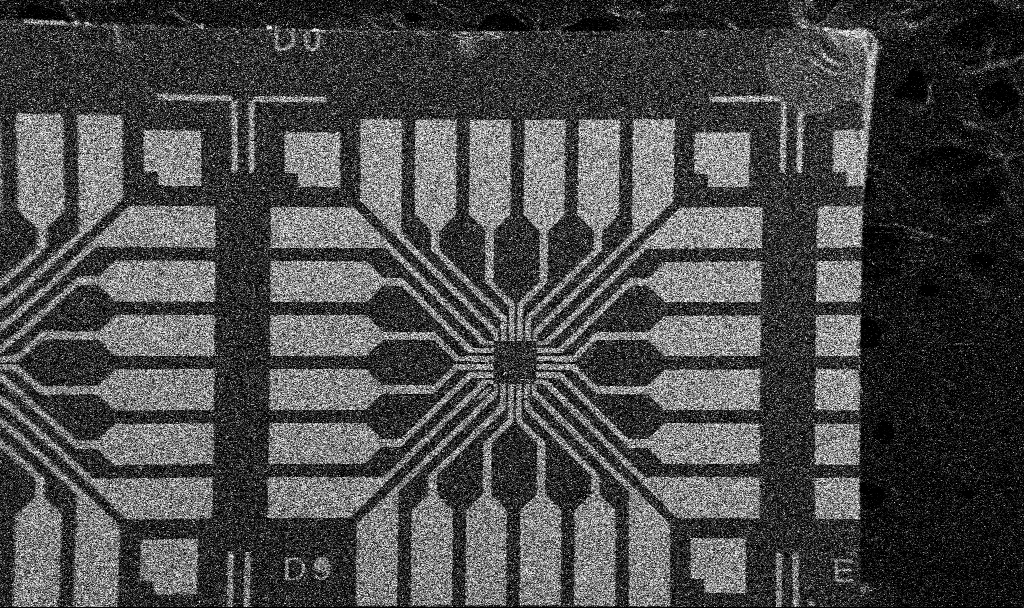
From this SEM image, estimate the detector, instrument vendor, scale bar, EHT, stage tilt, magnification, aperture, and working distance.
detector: SE2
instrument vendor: Zeiss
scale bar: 200000 nm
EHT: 5 kV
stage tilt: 0°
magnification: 0.1 K X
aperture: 30 µm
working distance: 10.7 mm